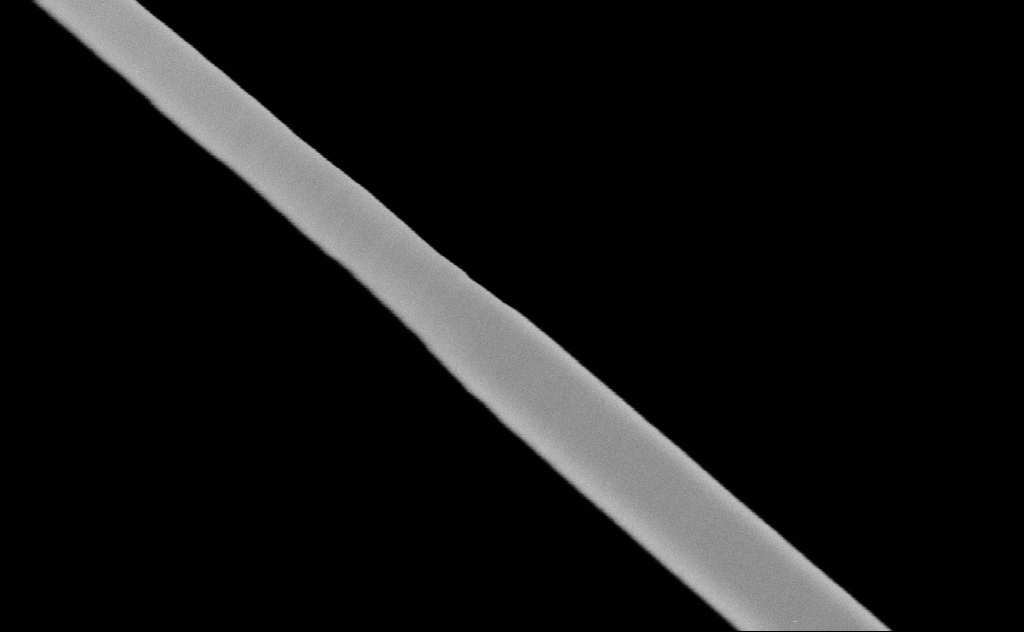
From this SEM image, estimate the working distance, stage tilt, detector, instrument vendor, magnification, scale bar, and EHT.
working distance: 10 mm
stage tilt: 0°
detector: SE2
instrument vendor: Zeiss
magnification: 245.42 K X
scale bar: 200 nm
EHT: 20 kV